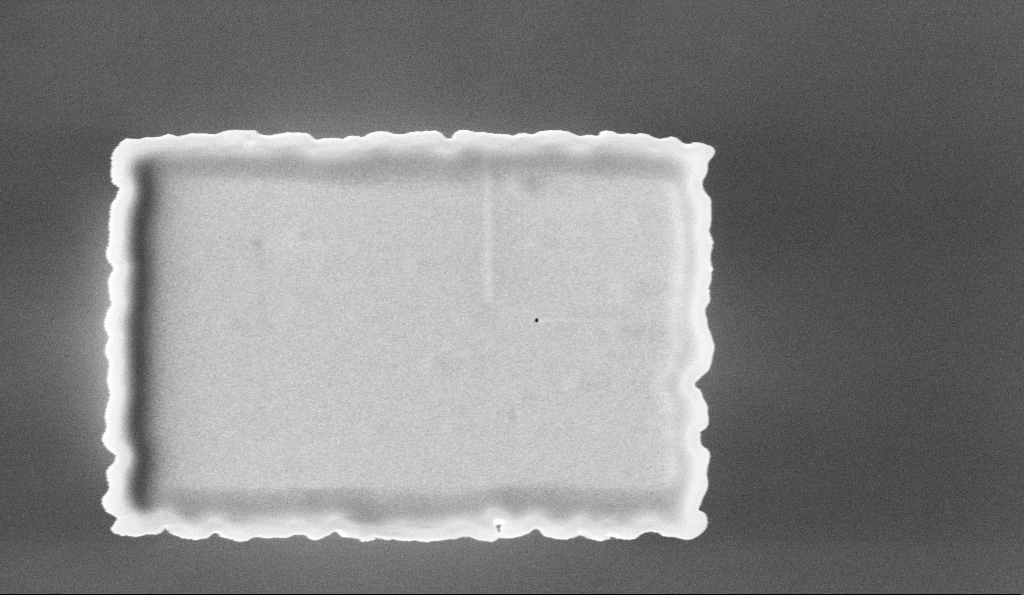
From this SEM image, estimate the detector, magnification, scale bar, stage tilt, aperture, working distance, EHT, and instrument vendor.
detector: InLens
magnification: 49.46 K X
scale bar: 1000 nm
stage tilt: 0°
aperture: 30 µm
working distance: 5.6 mm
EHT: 5 kV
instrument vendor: Zeiss